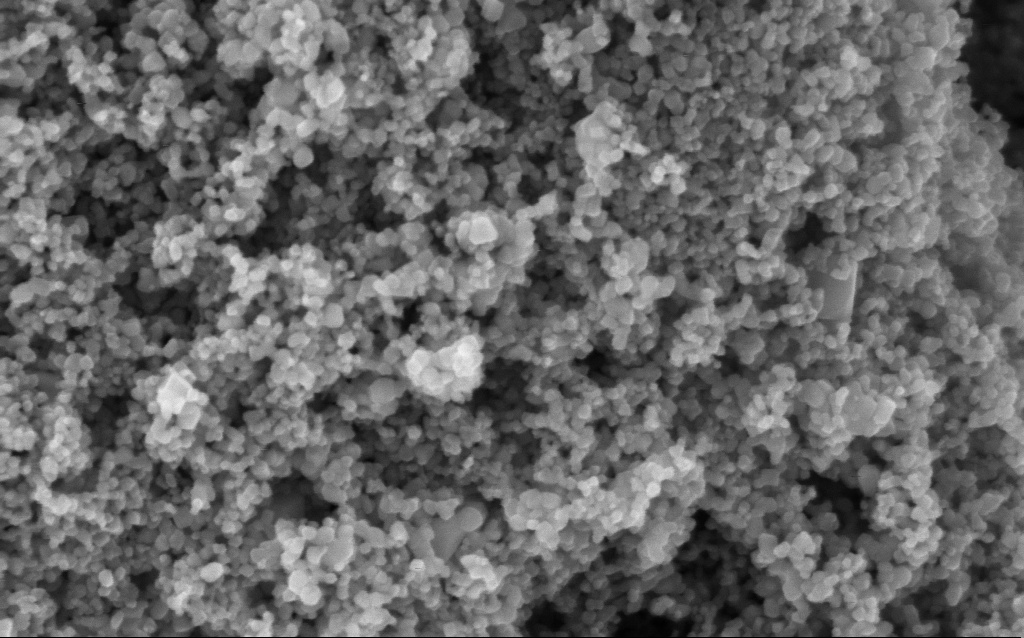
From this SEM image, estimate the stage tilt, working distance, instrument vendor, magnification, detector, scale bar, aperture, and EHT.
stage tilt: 0°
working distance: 4.6 mm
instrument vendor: Zeiss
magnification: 176.48 K X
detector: InLens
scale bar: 100 nm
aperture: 30 µm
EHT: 5 kV